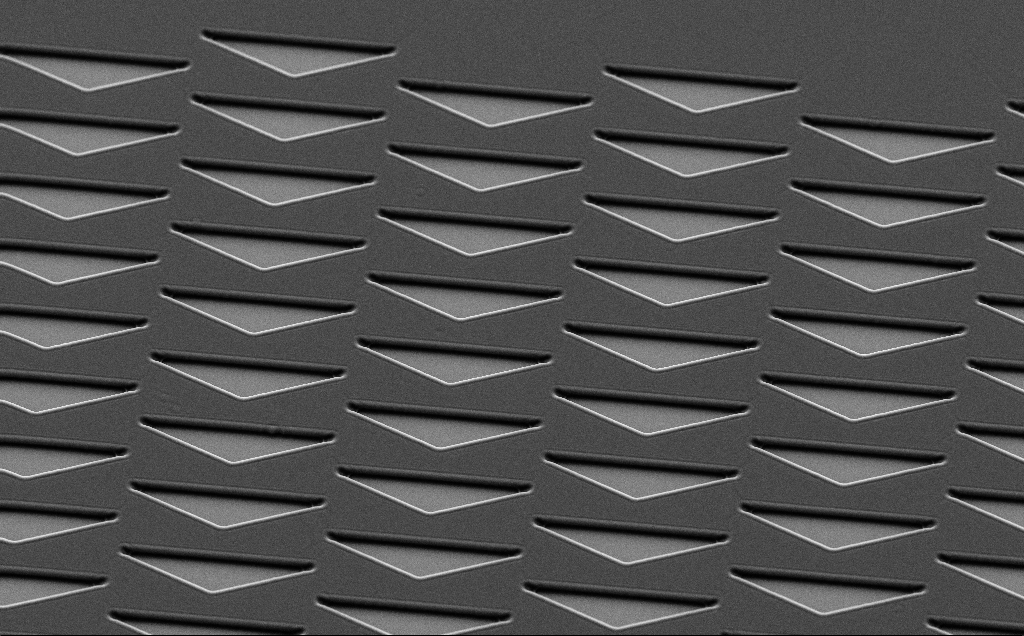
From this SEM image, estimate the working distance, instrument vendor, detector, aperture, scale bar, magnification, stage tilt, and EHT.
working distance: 5 mm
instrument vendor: Zeiss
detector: SE2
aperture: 30 µm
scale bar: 10000 nm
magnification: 1.77 K X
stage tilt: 35°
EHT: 7 kV